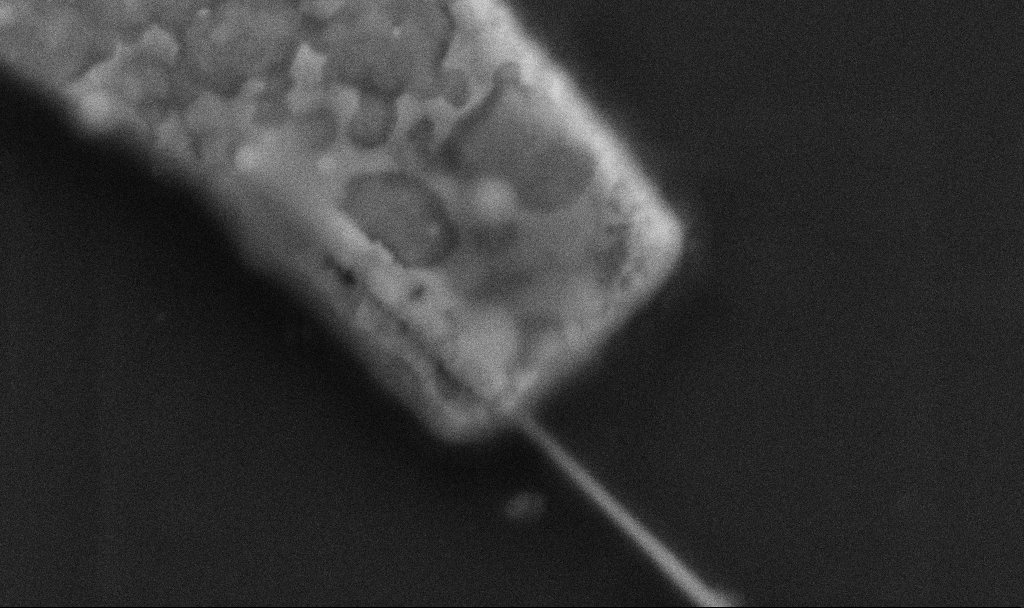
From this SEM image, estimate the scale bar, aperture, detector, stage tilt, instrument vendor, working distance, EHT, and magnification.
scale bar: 200 nm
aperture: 30 µm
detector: SE2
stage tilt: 0°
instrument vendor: Zeiss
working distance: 8.5 mm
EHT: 5 kV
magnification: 100 K X